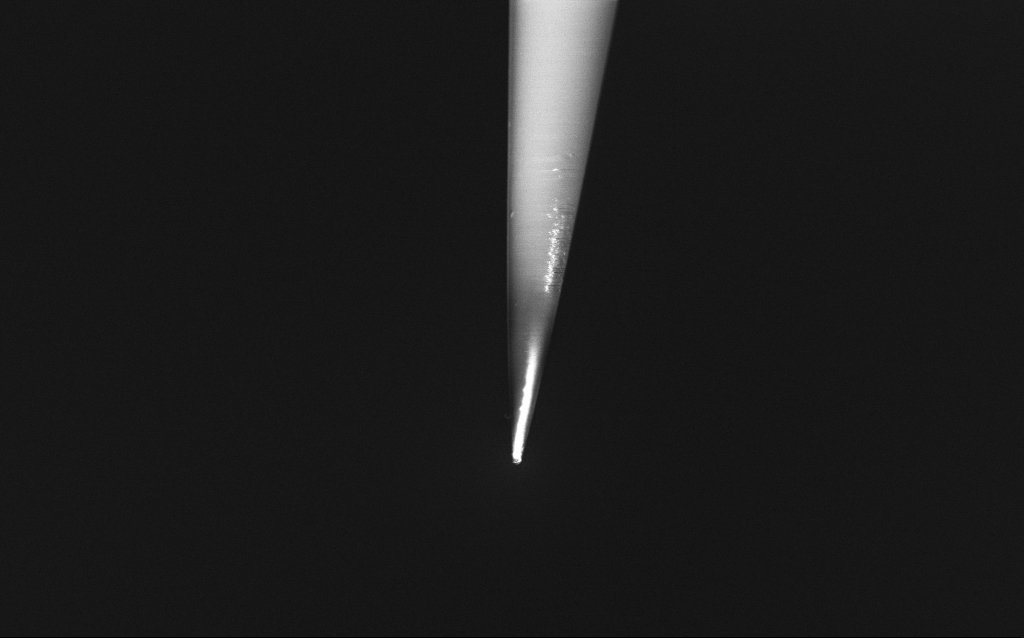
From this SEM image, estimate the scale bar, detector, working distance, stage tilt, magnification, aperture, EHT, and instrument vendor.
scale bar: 2000 nm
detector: InLens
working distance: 6 mm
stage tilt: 45°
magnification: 10 K X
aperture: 30 µm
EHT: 2 kV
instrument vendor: Zeiss